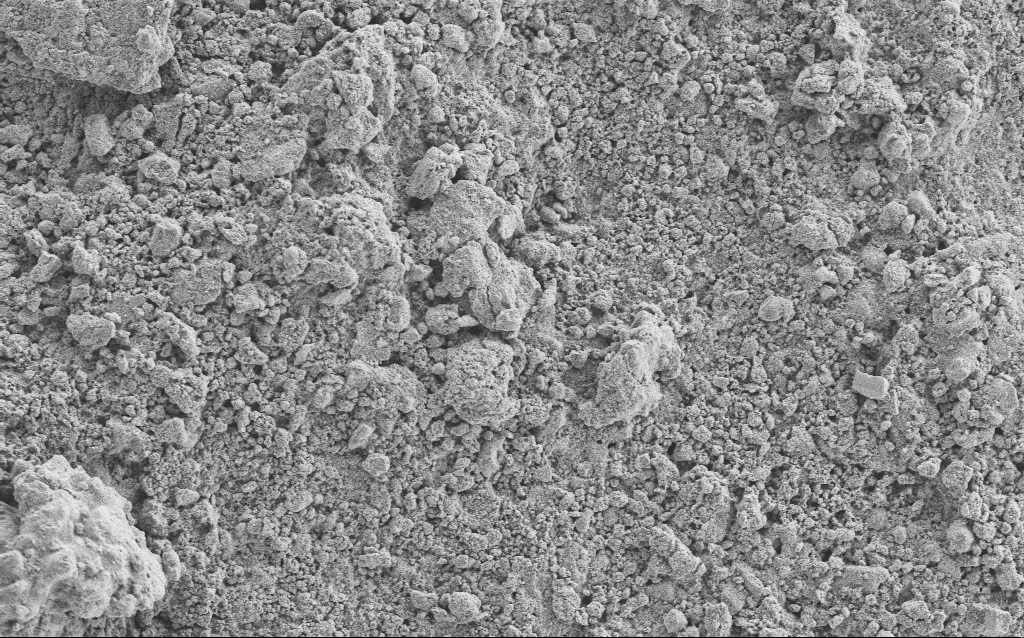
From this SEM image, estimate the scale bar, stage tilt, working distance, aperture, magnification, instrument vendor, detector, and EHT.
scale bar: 10000 nm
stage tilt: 0°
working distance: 4.6 mm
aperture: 30 µm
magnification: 1.23 K X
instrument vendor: Zeiss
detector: SE2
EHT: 5 kV